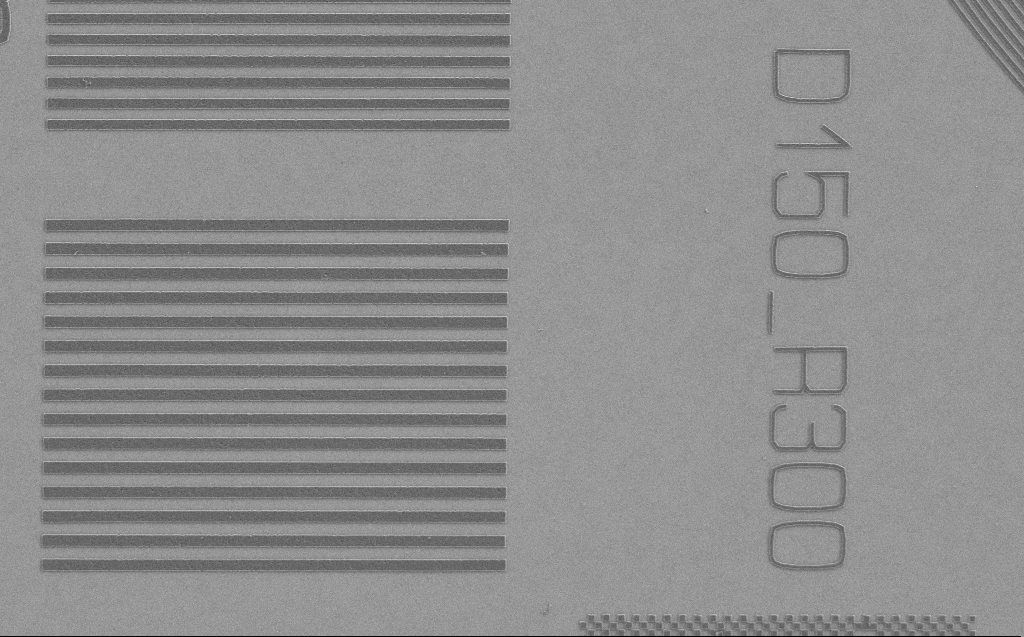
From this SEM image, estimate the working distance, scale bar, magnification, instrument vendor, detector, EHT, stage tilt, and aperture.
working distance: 5 mm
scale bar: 10000 nm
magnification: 5.63 K X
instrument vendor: Zeiss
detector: SE2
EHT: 1.2 kV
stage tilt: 0°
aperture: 30 µm